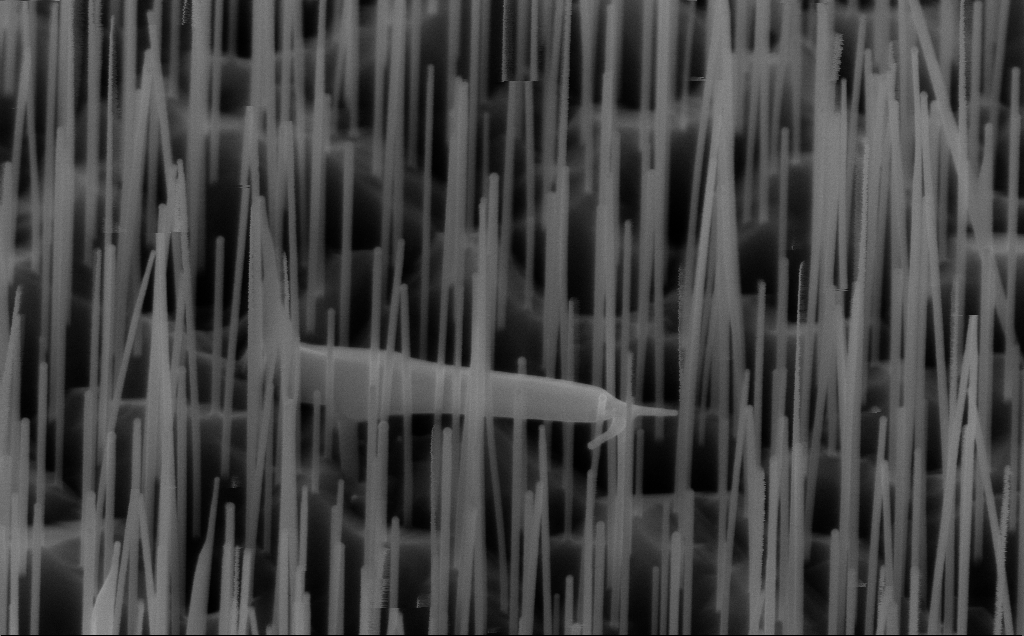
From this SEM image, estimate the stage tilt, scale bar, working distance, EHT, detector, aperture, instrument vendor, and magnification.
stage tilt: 45°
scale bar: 200 nm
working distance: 6 mm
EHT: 10 kV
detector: InLens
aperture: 30 µm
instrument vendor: Zeiss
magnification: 80 K X